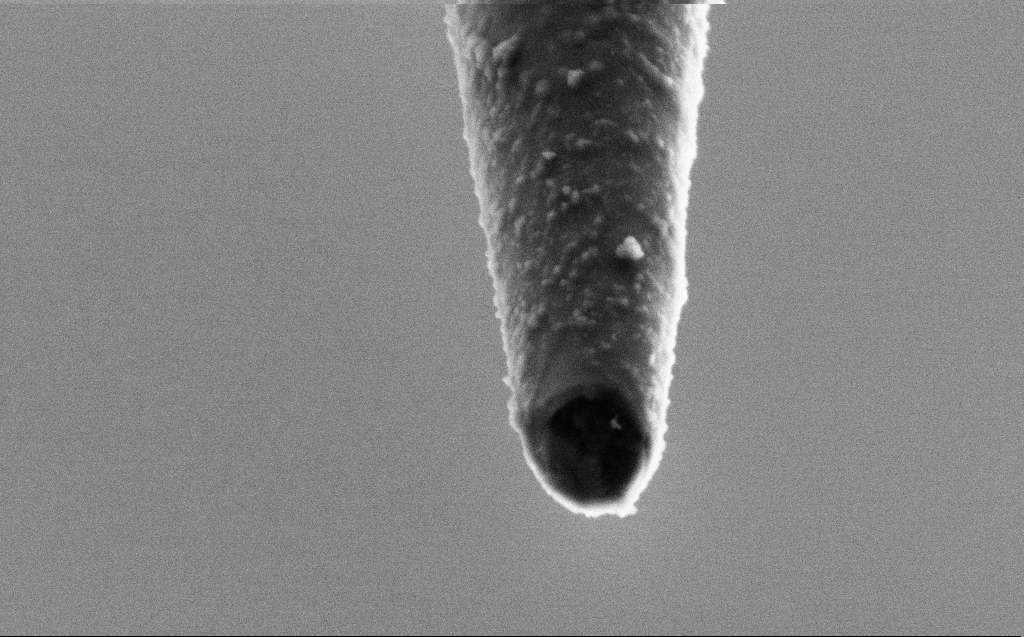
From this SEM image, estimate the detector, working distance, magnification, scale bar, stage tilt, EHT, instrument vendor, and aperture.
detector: SE2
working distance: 4 mm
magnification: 100 K X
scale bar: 200 nm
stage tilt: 45°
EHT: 2 kV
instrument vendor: Zeiss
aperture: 30 µm